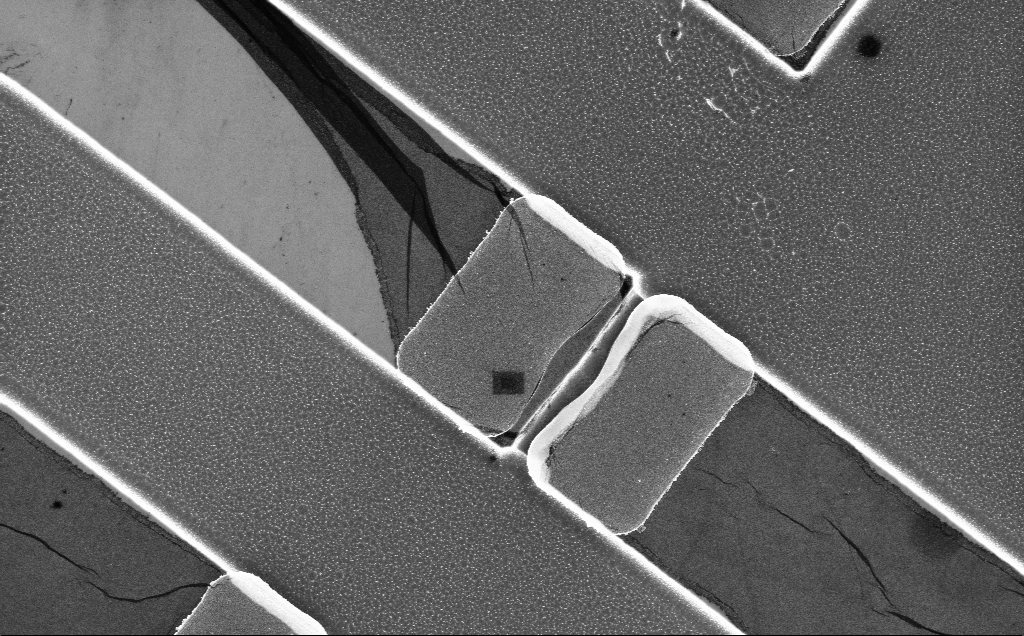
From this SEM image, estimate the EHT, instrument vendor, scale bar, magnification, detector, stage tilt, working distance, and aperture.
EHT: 5 kV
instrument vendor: Zeiss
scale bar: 10000 nm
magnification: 4.42 K X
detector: InLens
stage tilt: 0°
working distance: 10 mm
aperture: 30 µm